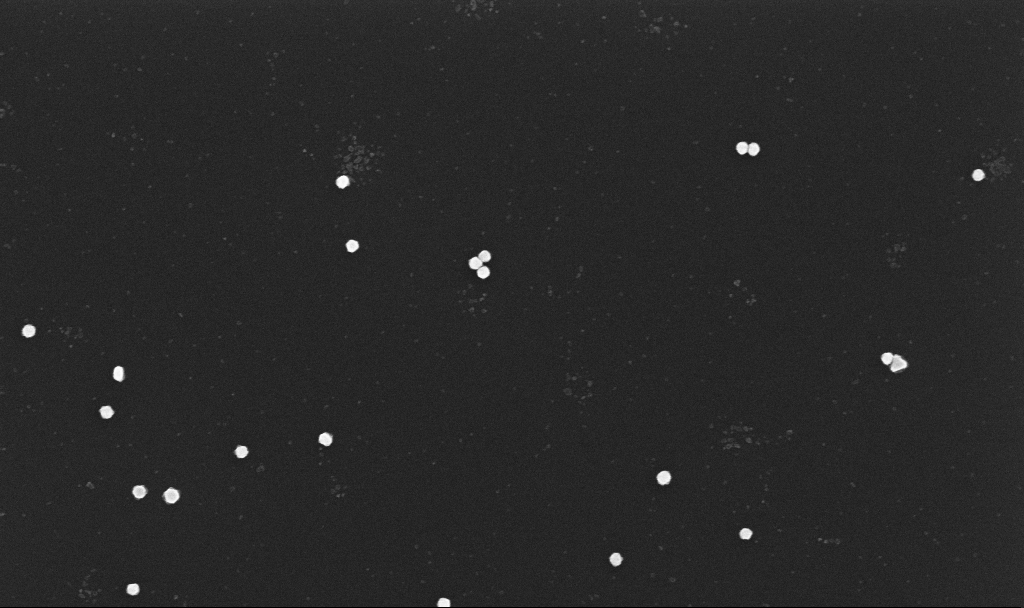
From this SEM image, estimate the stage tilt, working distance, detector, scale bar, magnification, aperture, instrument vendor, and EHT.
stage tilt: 0°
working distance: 3.3 mm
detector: InLens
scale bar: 1000 nm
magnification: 70 K X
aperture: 30 µm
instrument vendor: Zeiss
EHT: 10 kV